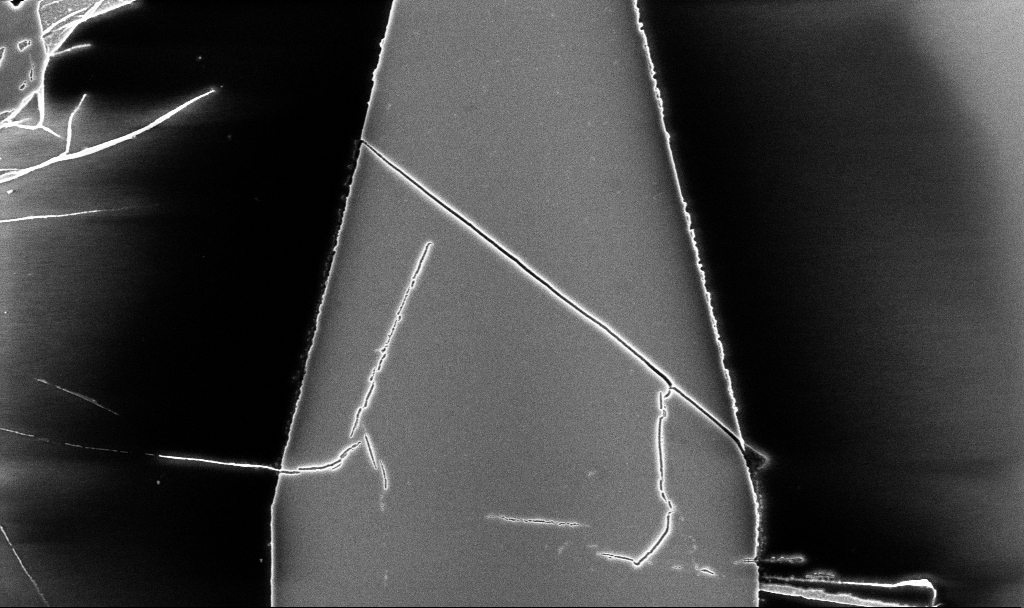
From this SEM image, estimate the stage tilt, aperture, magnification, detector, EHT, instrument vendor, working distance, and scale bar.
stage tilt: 0°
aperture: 30 µm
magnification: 9.09 K X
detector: InLens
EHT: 5 kV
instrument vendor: Zeiss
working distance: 5.2 mm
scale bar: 2000 nm